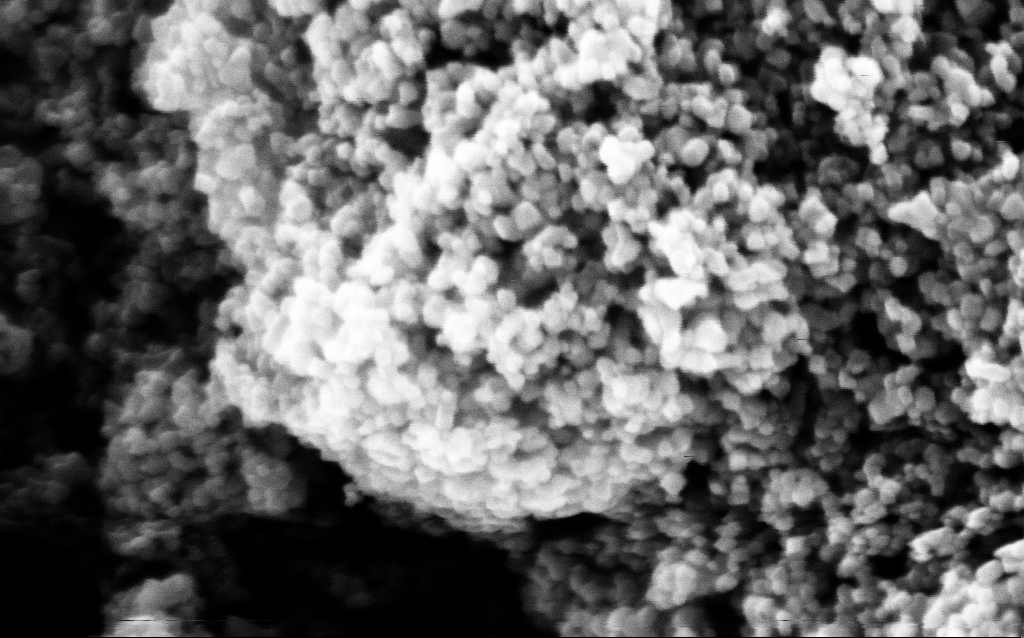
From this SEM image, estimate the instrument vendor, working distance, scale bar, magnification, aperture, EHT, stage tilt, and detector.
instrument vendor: Zeiss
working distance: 1.6 mm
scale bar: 200 nm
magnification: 274.78 K X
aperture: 30 µm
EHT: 5 kV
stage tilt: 0°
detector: InLens